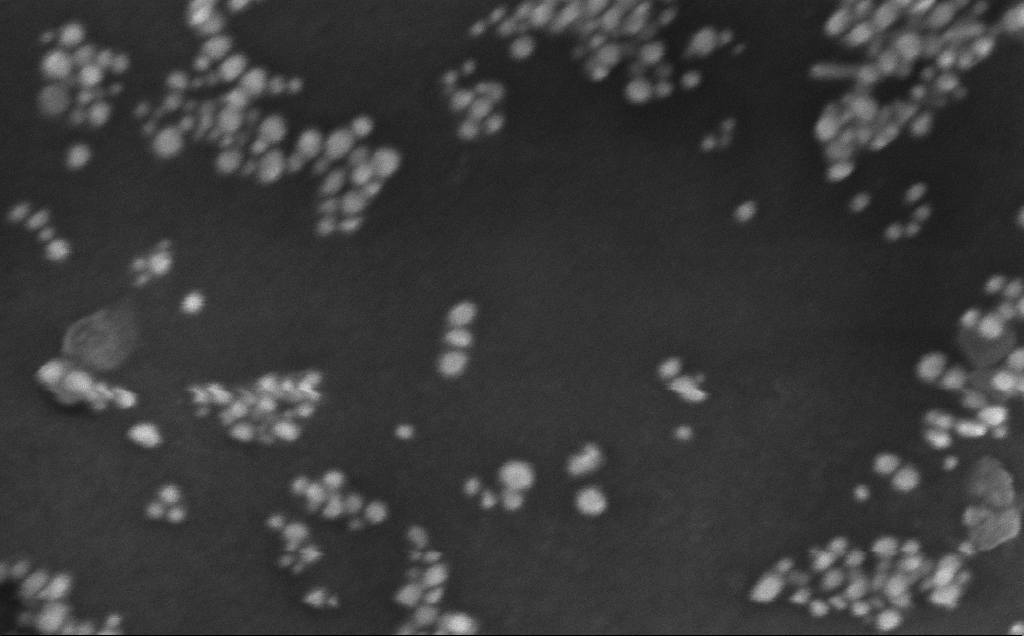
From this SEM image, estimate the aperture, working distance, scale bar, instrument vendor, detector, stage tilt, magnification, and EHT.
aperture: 30 µm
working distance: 4 mm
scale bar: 200 nm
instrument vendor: Zeiss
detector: InLens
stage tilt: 0°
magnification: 362.43 K X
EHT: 10 kV